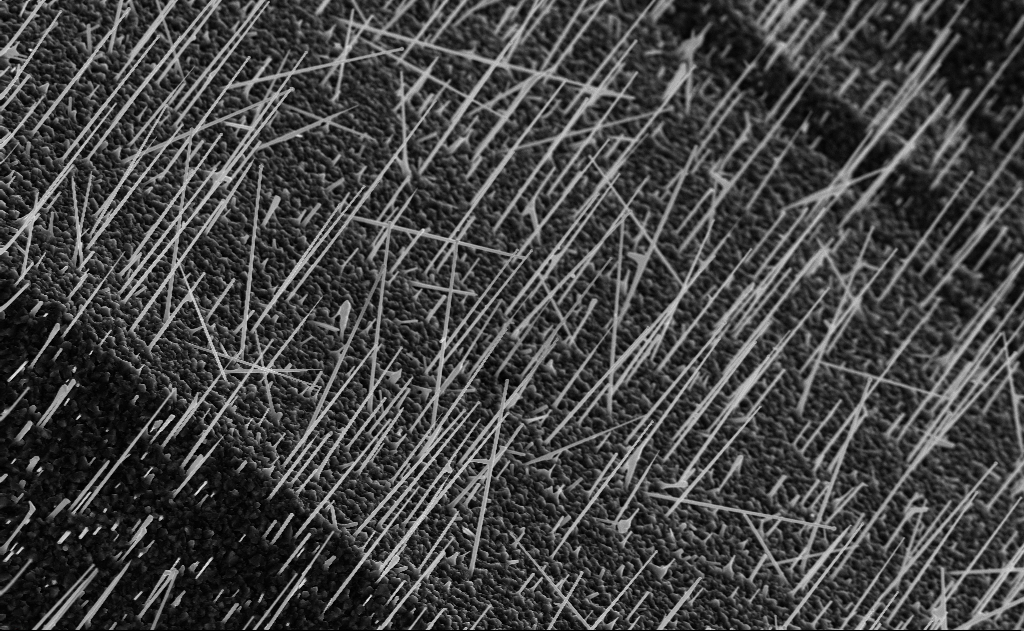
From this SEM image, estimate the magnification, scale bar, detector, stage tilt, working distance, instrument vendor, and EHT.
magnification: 10 K X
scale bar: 2000 nm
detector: InLens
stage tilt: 0°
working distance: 10 mm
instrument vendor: Zeiss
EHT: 10 kV